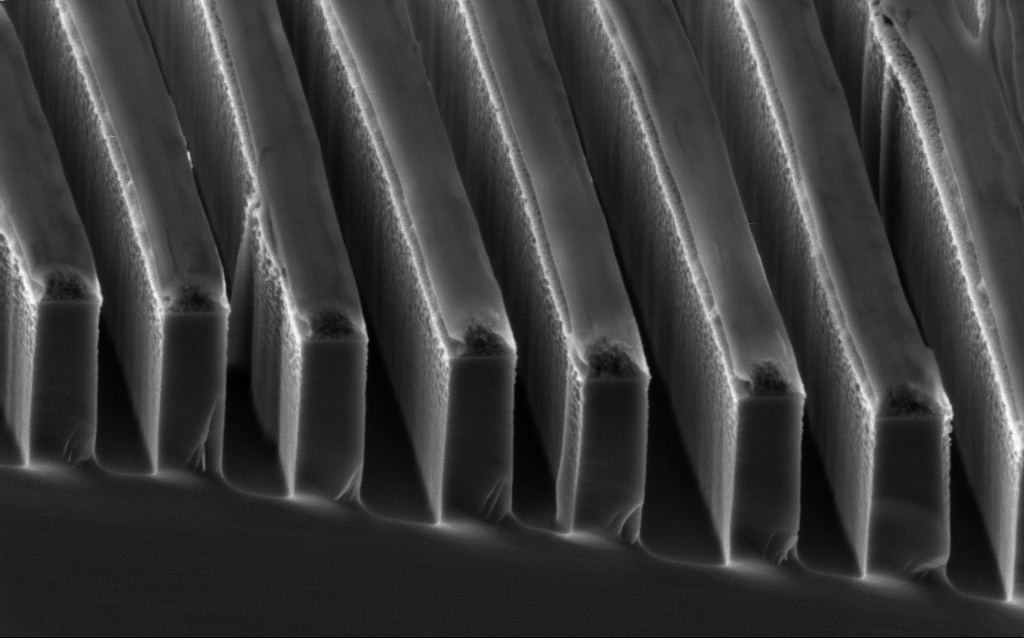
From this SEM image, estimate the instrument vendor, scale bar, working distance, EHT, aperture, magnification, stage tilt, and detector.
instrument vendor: Zeiss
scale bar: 200 nm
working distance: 3.6 mm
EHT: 2 kV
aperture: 30 µm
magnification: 94.43 K X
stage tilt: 45°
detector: InLens